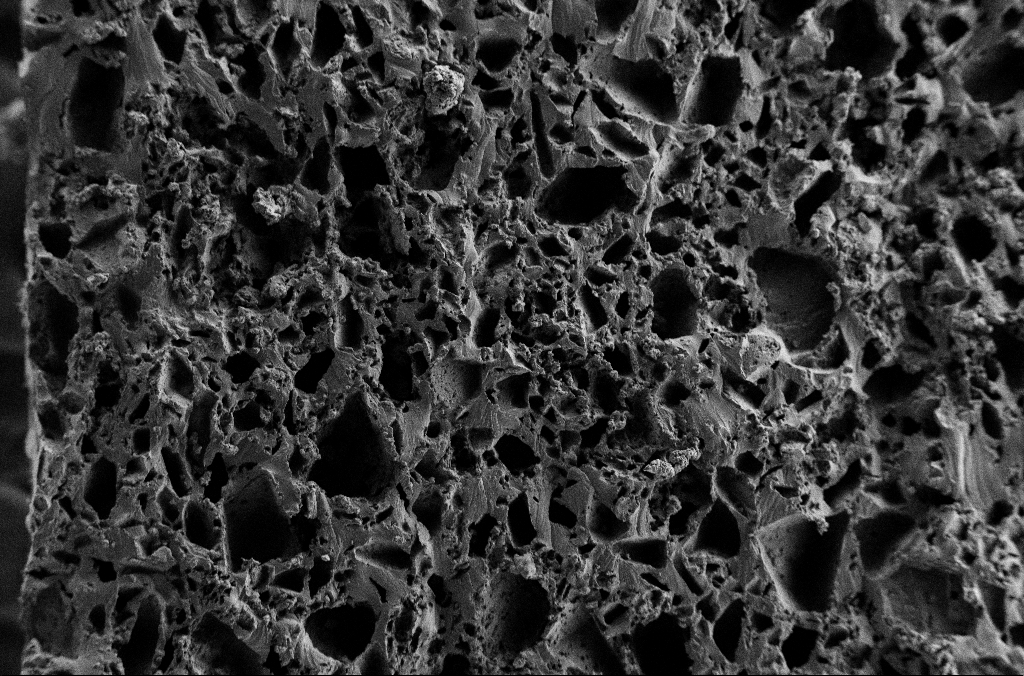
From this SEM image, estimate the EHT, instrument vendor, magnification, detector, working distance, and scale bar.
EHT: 2 kV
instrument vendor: Zeiss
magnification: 0.2 K X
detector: SE2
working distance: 2.9 mm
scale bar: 100000 nm